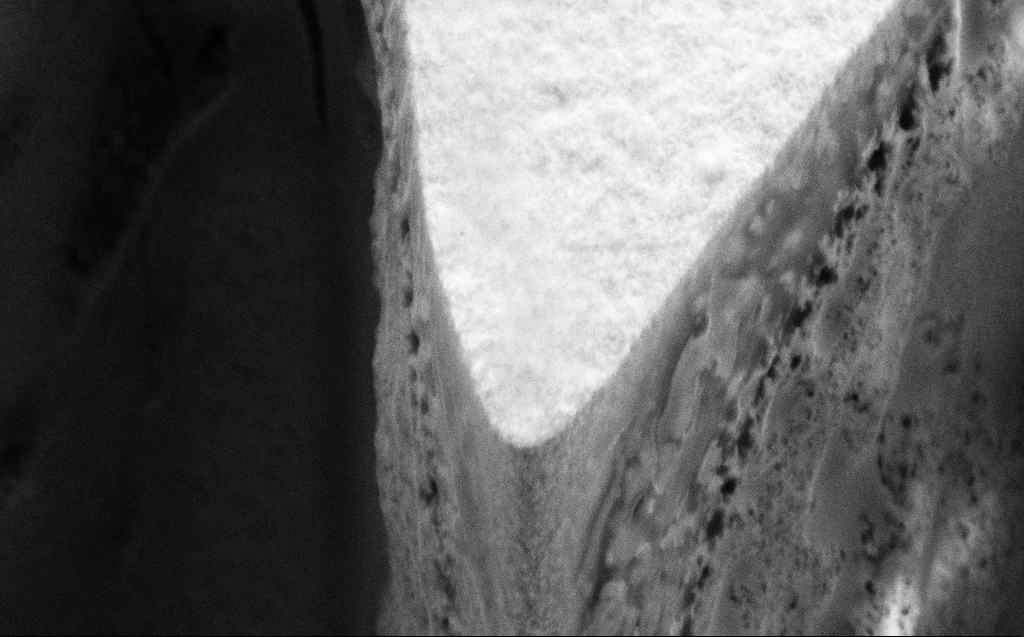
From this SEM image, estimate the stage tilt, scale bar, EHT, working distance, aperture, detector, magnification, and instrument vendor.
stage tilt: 45°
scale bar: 1000 nm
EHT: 5 kV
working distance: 7 mm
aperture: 30 µm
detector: SE2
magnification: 37.2 K X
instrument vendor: Zeiss